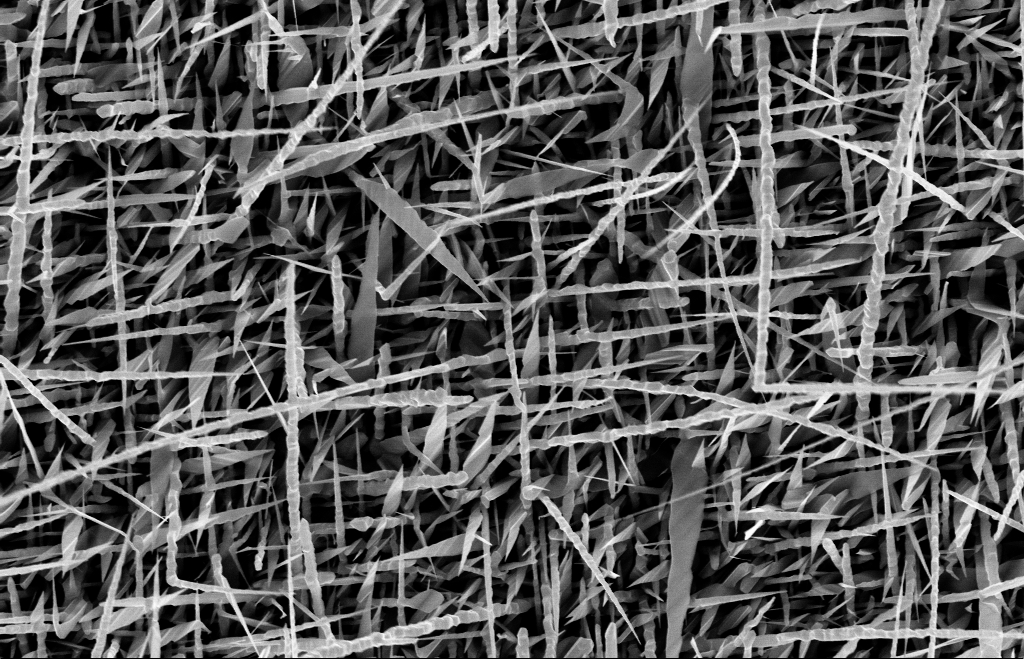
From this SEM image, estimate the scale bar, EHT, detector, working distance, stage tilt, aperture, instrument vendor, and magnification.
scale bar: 1000 nm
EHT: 10 kV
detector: InLens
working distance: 9 mm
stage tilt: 0°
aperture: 30 µm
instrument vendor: Zeiss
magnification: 20 K X